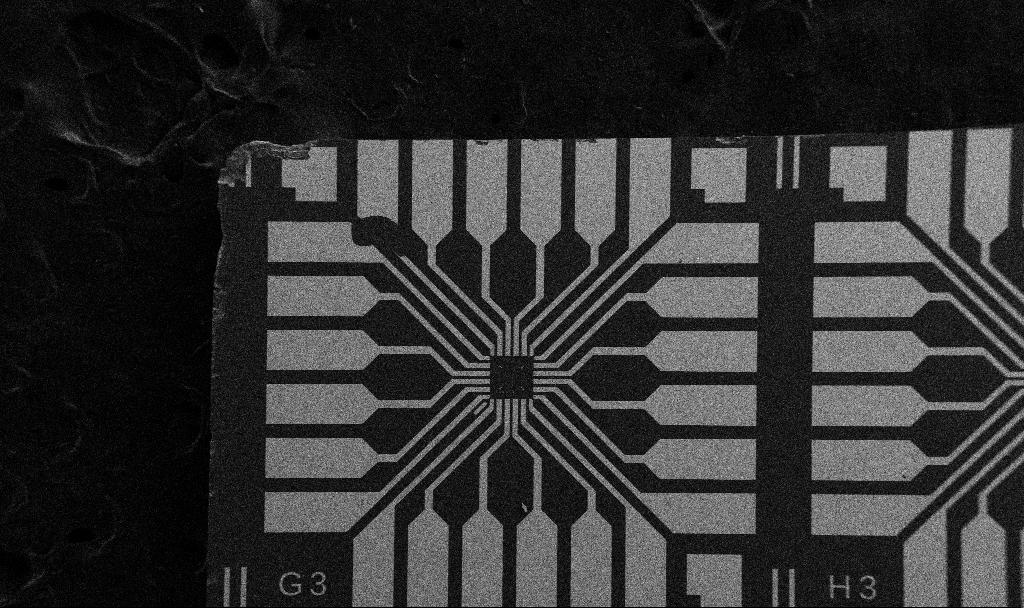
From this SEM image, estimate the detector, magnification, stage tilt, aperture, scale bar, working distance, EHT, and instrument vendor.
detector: SE2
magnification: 0.1 K X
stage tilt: -0°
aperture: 30 µm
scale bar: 200000 nm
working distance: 10.7 mm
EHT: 5 kV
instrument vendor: Zeiss